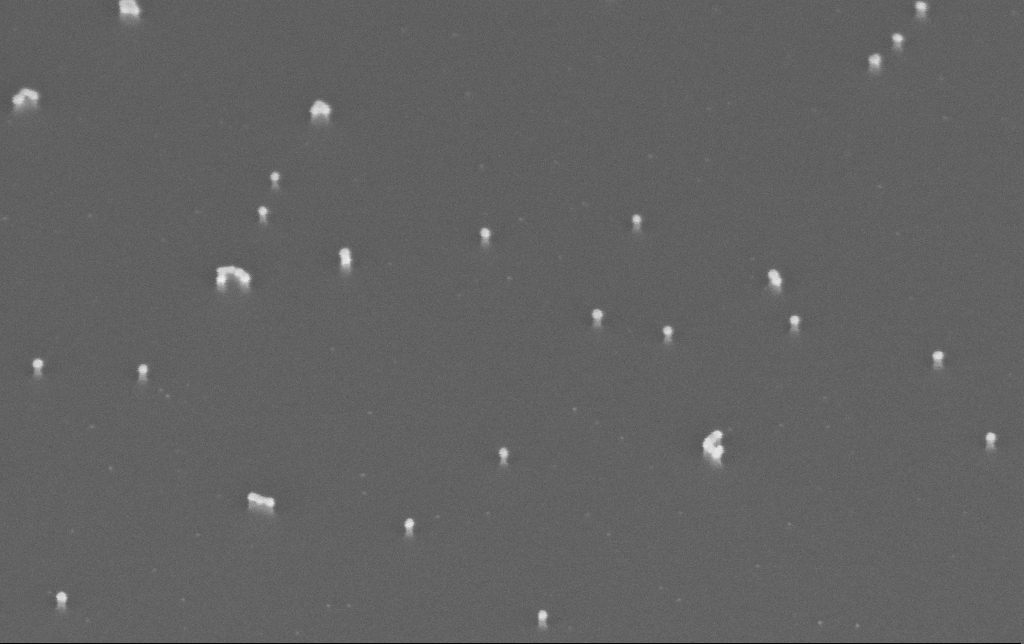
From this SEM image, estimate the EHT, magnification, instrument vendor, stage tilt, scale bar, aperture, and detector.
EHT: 10 kV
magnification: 200 K X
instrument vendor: Zeiss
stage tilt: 45°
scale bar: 100 nm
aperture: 30 µm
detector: InLens